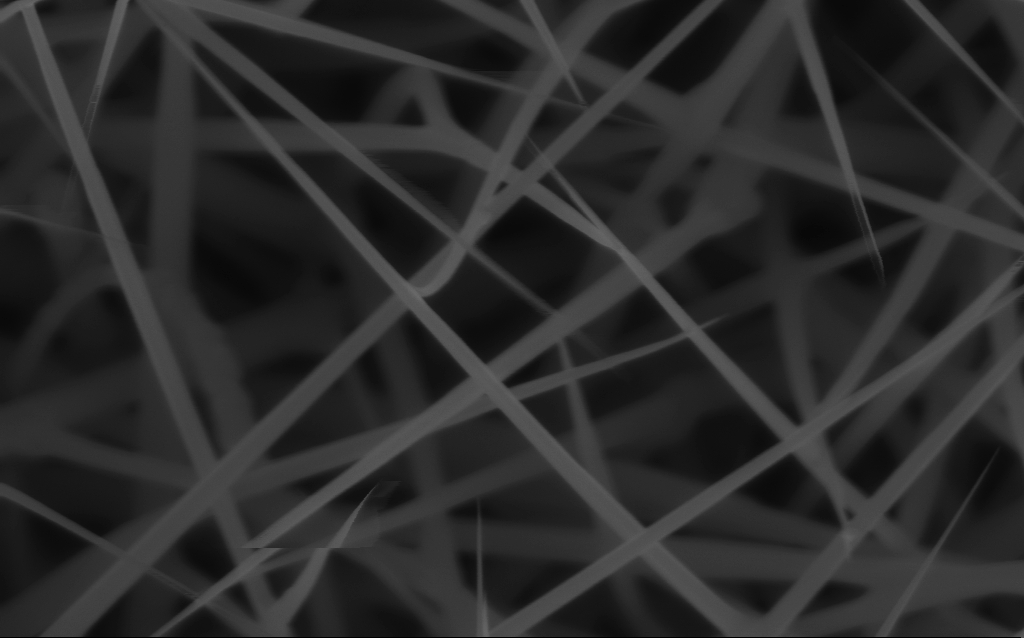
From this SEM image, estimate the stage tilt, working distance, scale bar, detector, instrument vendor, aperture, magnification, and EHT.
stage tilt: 0°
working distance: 6 mm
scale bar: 200 nm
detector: InLens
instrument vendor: Zeiss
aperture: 30 µm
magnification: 80 K X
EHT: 10 kV